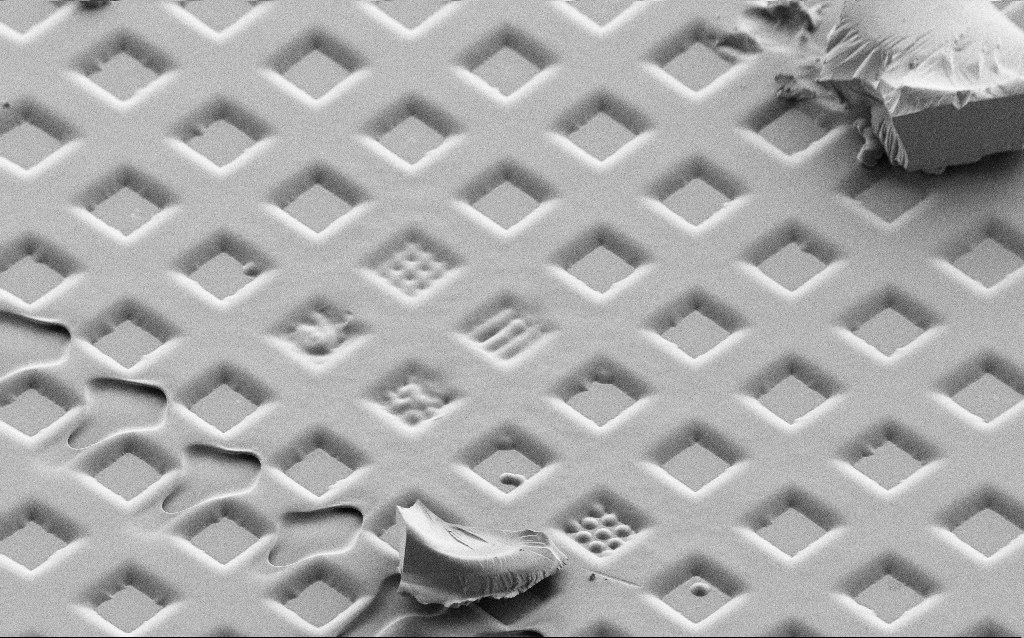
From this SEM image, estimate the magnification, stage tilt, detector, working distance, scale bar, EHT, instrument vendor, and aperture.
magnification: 0.345 K X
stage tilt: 45°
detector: SE2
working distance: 6 mm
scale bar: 100000 nm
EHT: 1.5 kV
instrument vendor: Zeiss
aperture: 30 µm